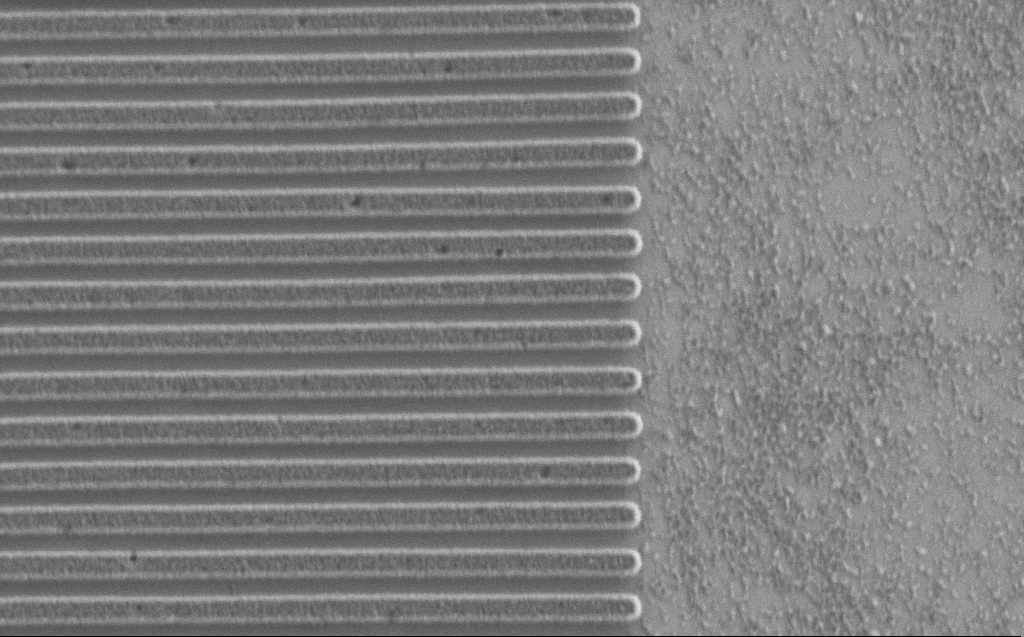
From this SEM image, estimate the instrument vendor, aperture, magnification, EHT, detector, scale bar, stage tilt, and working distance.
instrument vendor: Zeiss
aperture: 30 µm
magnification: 41.66 K X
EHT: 1.2 kV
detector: SE2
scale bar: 1000 nm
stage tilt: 0°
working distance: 5 mm